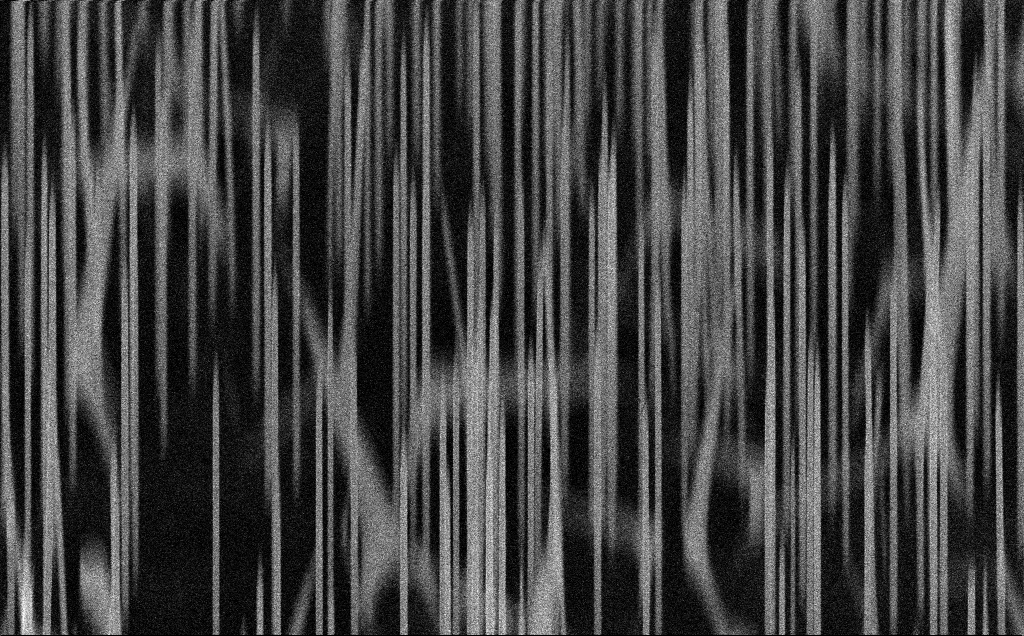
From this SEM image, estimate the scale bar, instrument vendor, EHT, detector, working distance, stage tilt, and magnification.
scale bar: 1000 nm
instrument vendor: Zeiss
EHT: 10 kV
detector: InLens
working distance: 9 mm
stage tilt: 45°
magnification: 43.9 K X